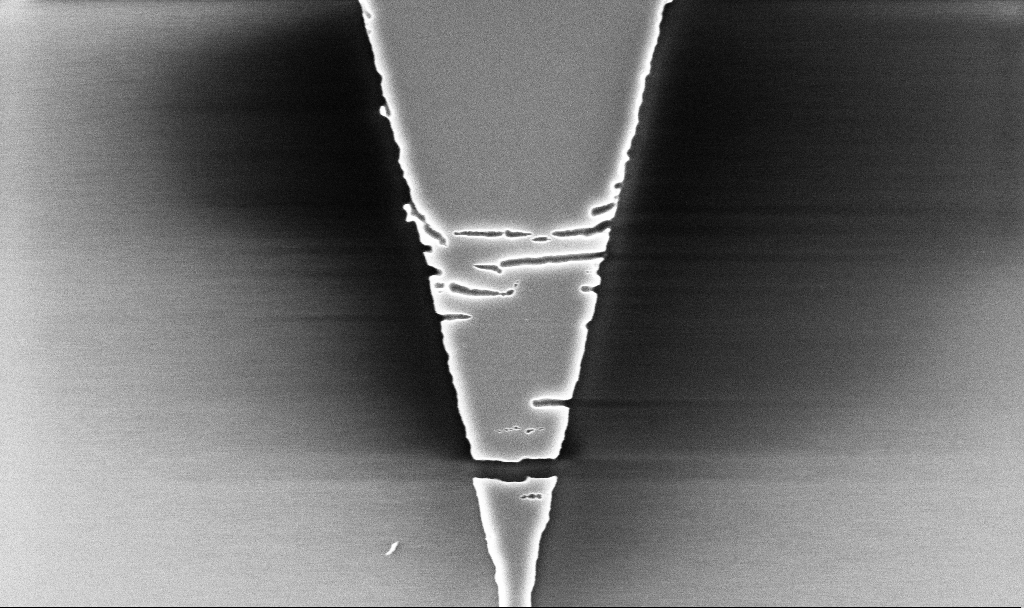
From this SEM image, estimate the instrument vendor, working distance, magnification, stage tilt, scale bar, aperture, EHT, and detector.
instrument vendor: Zeiss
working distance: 5.2 mm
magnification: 22.46 K X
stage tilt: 0°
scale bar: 2000 nm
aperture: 30 µm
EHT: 5 kV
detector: InLens